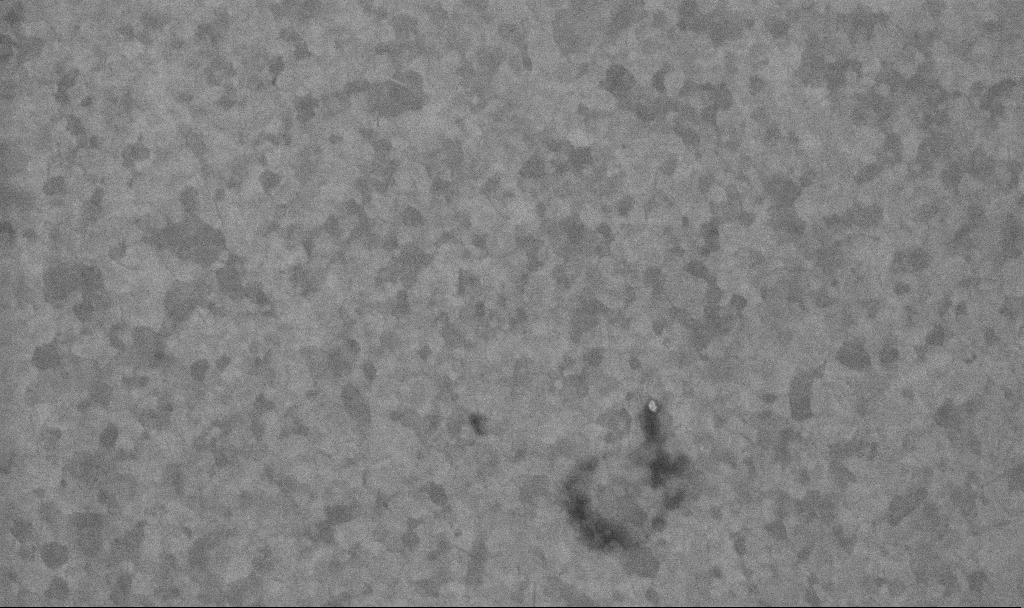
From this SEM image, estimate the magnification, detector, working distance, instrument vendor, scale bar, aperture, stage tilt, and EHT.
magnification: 100.94 K X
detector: InLens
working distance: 3.4 mm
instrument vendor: Zeiss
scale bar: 200 nm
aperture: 30 µm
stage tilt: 0°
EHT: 10 kV